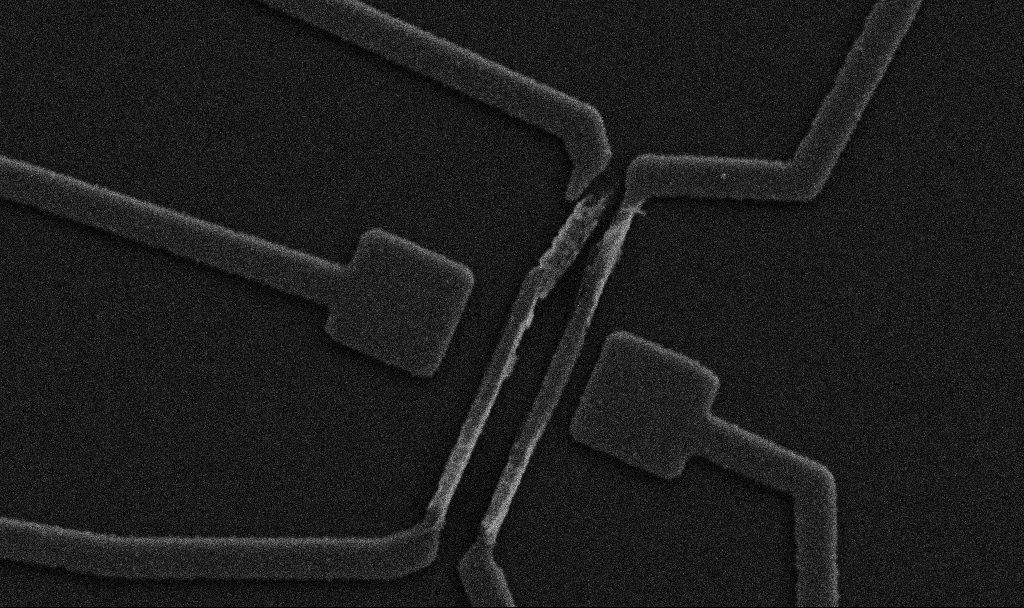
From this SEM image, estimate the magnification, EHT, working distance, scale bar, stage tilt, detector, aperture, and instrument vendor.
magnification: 20 K X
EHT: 5 kV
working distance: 10.7 mm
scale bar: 1000 nm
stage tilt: -0°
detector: SE2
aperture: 30 µm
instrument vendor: Zeiss